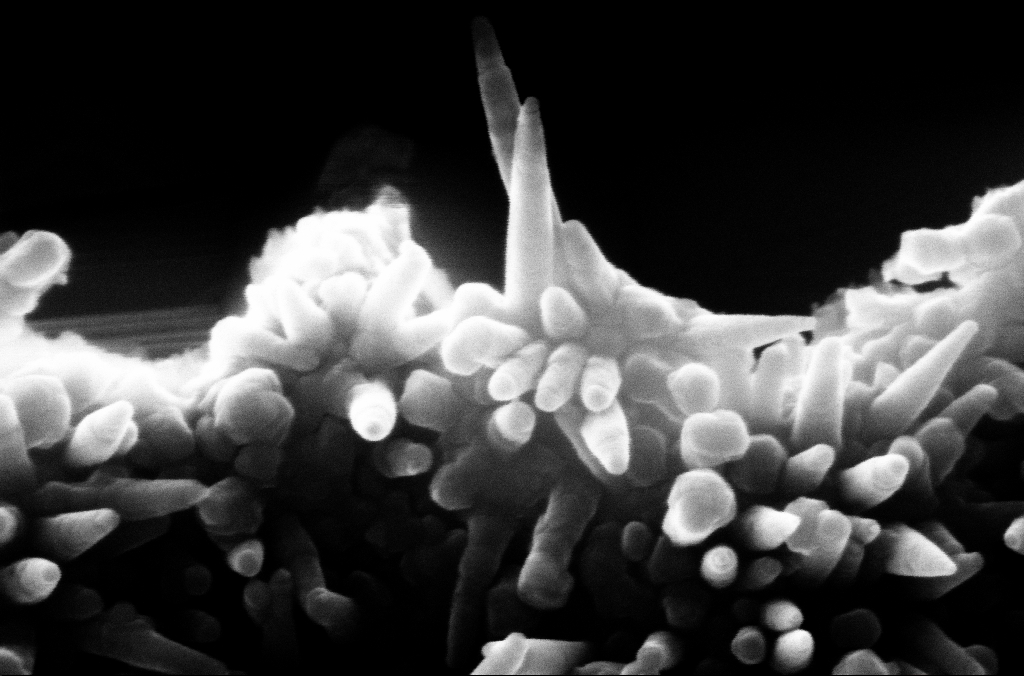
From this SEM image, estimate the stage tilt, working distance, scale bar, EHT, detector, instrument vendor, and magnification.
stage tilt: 0.1°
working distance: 5.2 mm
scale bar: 100 nm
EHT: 10 kV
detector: InLens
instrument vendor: Zeiss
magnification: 279.37 K X